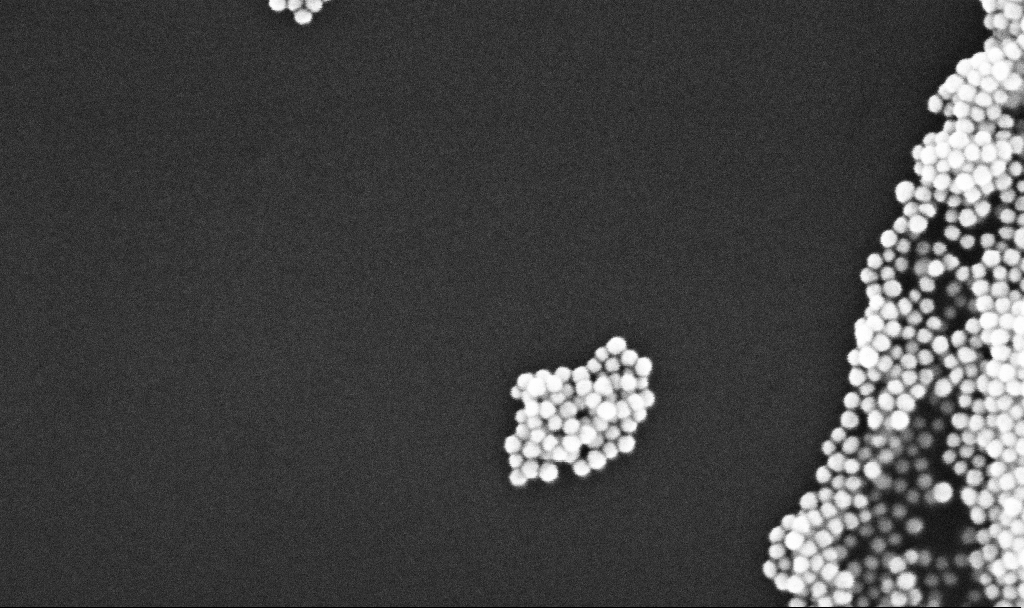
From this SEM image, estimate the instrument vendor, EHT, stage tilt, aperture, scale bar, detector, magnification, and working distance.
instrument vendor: Zeiss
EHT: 10 kV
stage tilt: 0°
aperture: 30 µm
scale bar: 200 nm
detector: InLens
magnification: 298.11 K X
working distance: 3.3 mm